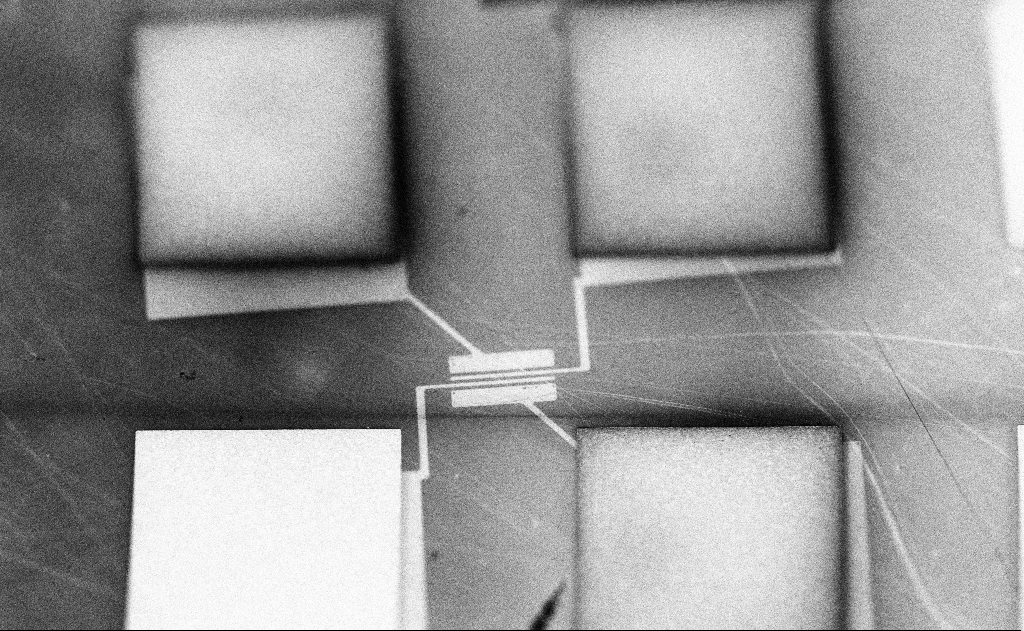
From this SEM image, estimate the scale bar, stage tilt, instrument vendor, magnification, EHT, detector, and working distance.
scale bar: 100000 nm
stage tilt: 0°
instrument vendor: Zeiss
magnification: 0.647 K X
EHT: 5 kV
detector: InLens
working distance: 10 mm